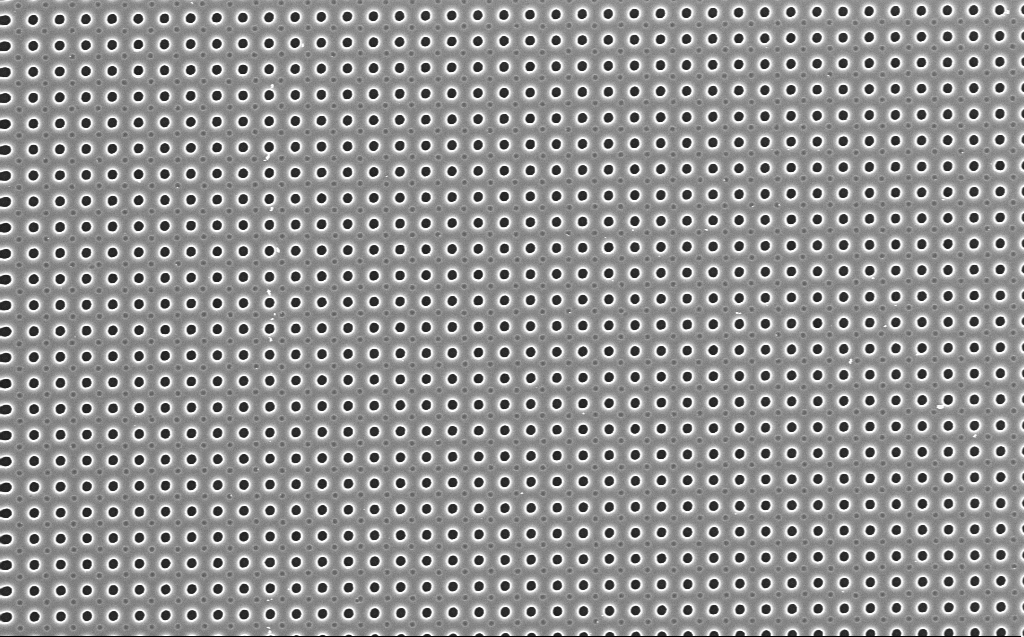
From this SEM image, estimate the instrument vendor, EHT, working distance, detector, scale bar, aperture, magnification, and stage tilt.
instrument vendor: Zeiss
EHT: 5 kV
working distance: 4 mm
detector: InLens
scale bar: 2000 nm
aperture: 30 µm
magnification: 9.2 K X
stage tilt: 0°